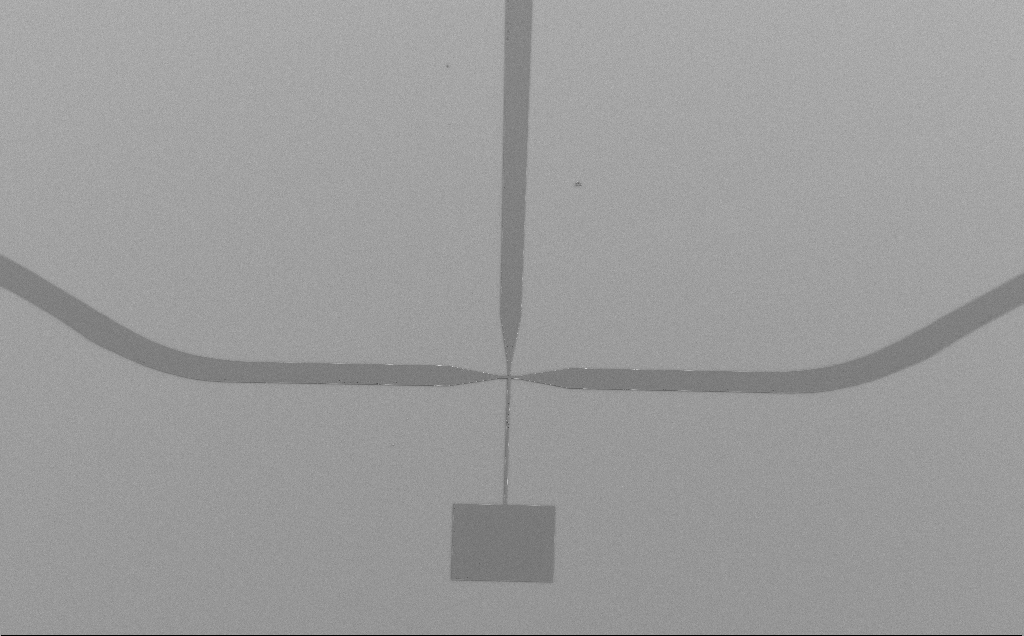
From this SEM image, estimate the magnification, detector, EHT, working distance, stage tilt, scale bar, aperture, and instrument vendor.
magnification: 0.094 K X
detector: SE2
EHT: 5 kV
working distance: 9 mm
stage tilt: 0°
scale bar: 200000 nm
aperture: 30 µm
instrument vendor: Zeiss